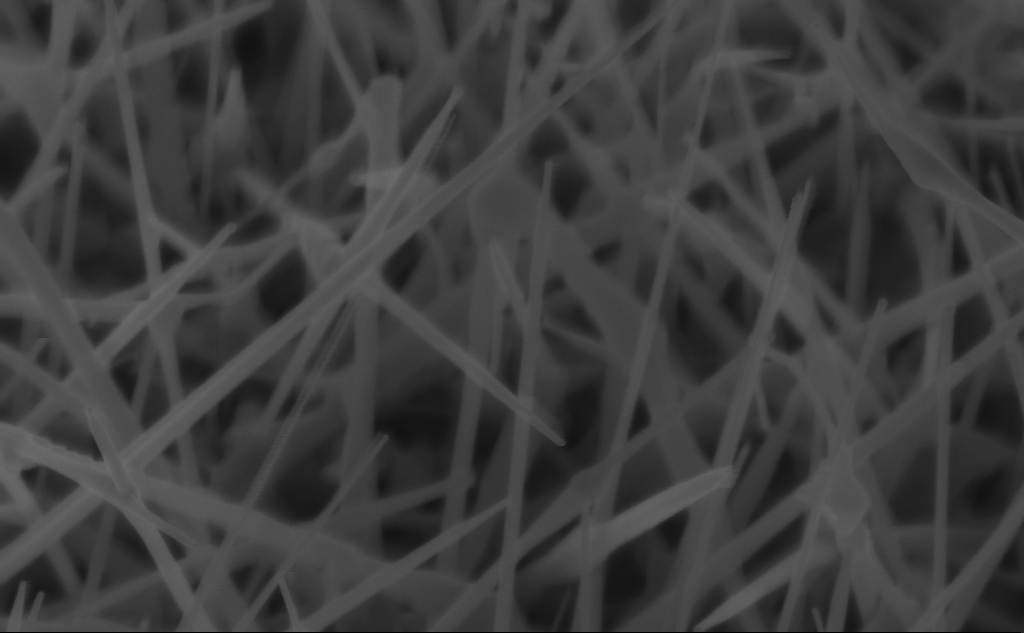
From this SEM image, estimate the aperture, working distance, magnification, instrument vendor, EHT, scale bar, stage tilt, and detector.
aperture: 30 µm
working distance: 4 mm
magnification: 104.11 K X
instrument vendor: Zeiss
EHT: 5 kV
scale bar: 200 nm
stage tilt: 45°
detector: InLens